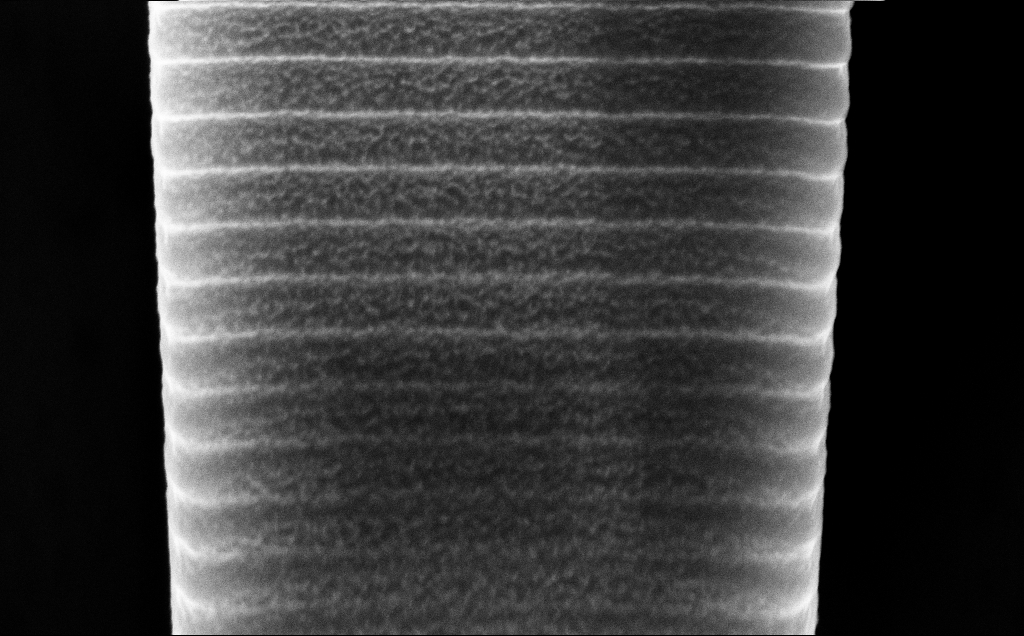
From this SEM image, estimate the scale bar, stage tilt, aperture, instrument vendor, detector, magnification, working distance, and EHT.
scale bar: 1000 nm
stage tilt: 45°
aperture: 30 µm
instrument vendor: Zeiss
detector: InLens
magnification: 56.33 K X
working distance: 5 mm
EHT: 10 kV